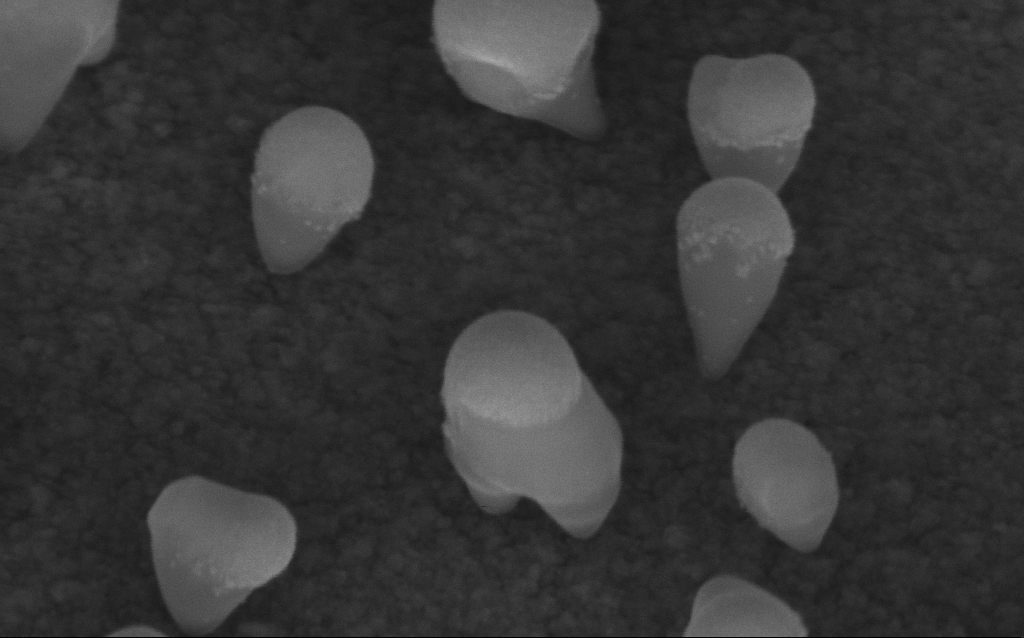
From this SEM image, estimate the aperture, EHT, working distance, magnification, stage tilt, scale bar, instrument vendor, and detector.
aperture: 30 µm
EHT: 5 kV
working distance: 6.4 mm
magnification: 290.69 K X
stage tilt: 45°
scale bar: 100 nm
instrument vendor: Zeiss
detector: InLens